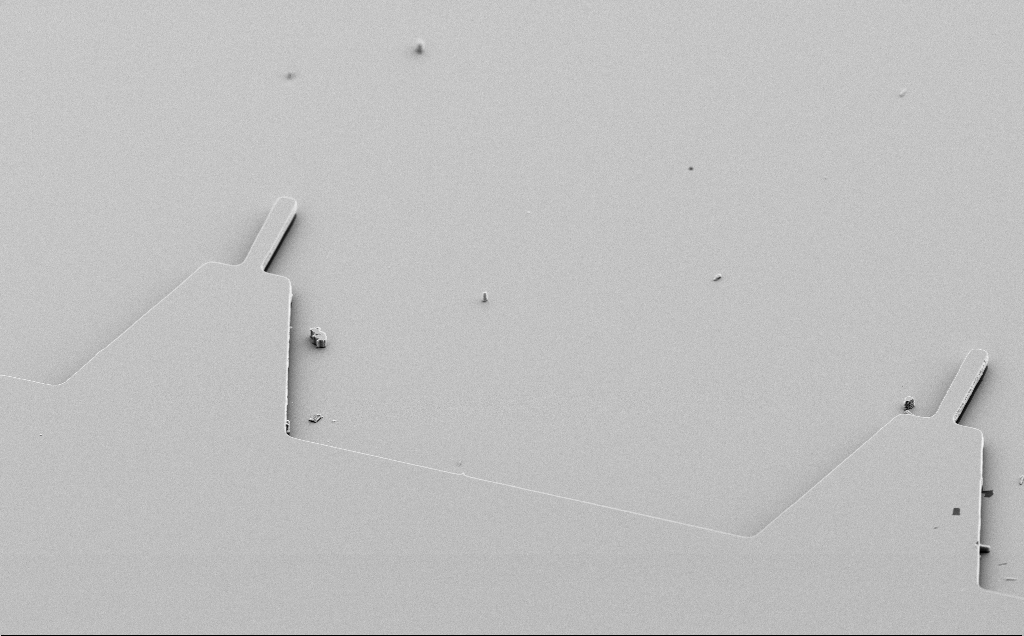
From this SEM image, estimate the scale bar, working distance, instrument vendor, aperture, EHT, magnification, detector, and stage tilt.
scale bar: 10000 nm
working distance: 10 mm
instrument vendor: Zeiss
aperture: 30 µm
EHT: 5 kV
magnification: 1.69 K X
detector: SE2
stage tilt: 50°